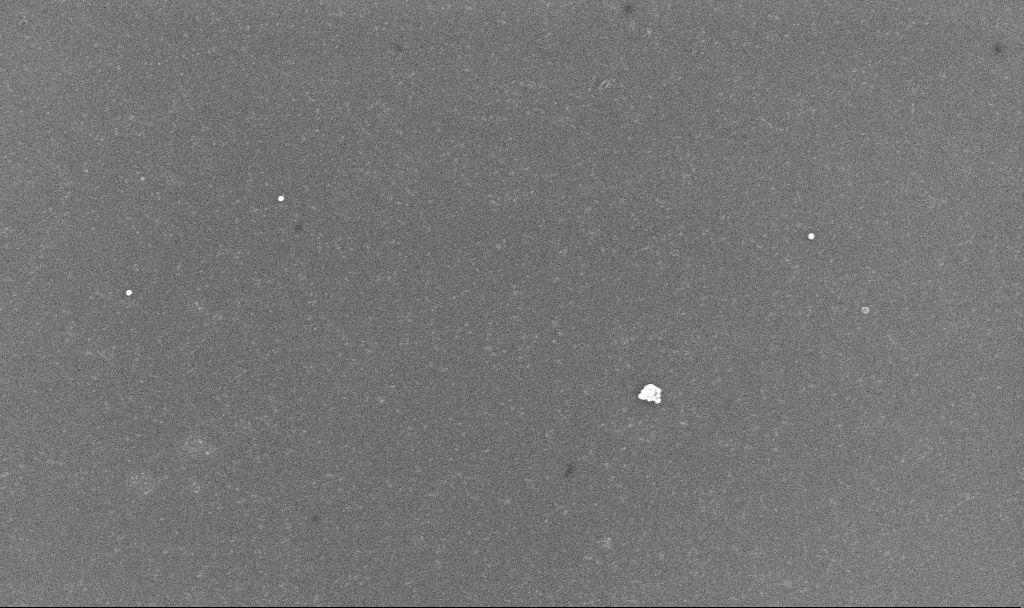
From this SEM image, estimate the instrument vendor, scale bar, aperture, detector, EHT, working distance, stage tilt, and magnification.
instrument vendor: Zeiss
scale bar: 200 nm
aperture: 30 µm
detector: InLens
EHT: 10 kV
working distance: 3.1 mm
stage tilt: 0°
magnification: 80 K X